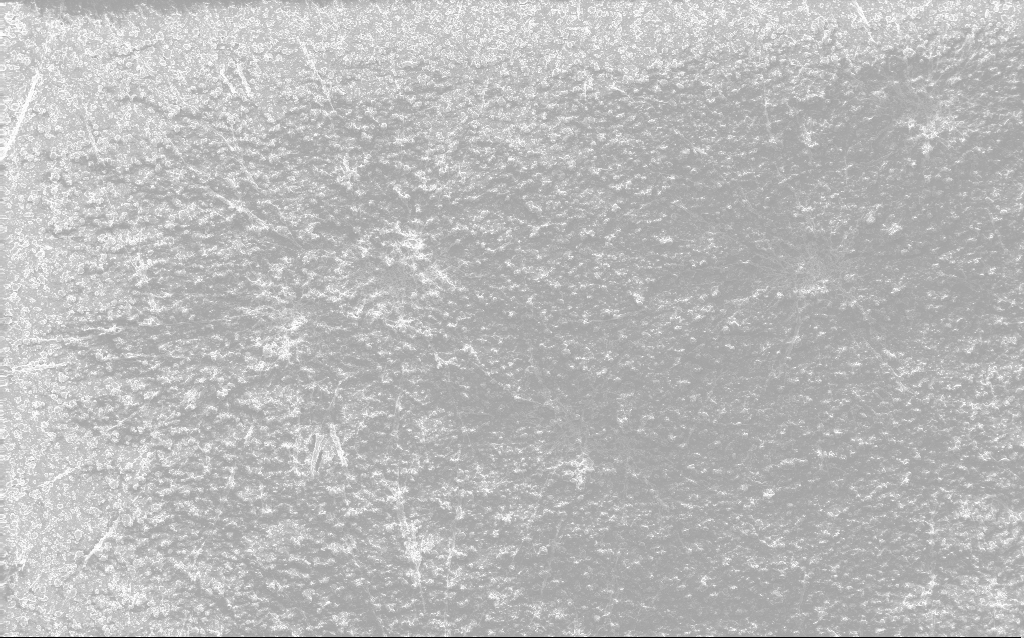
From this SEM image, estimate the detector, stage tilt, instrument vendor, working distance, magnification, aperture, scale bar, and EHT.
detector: InLens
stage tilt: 0°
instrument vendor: Zeiss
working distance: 2.7 mm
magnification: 0.235 K X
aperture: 30 µm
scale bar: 100000 nm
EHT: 10 kV